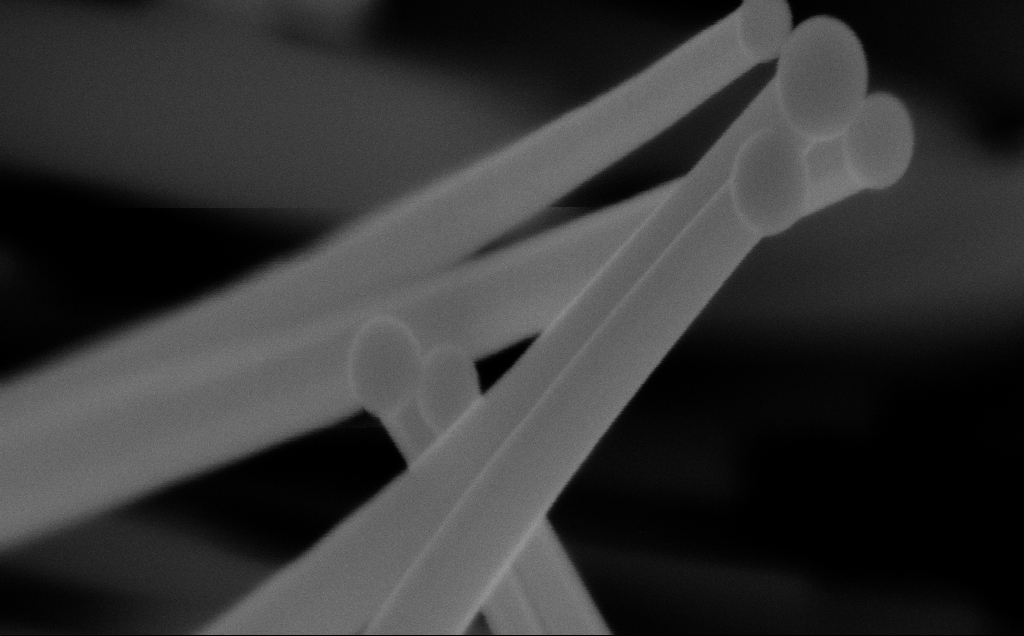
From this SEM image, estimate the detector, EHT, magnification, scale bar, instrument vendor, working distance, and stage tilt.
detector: InLens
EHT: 10 kV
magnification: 315.92 K X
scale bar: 200 nm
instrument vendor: Zeiss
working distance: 6 mm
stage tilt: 0°